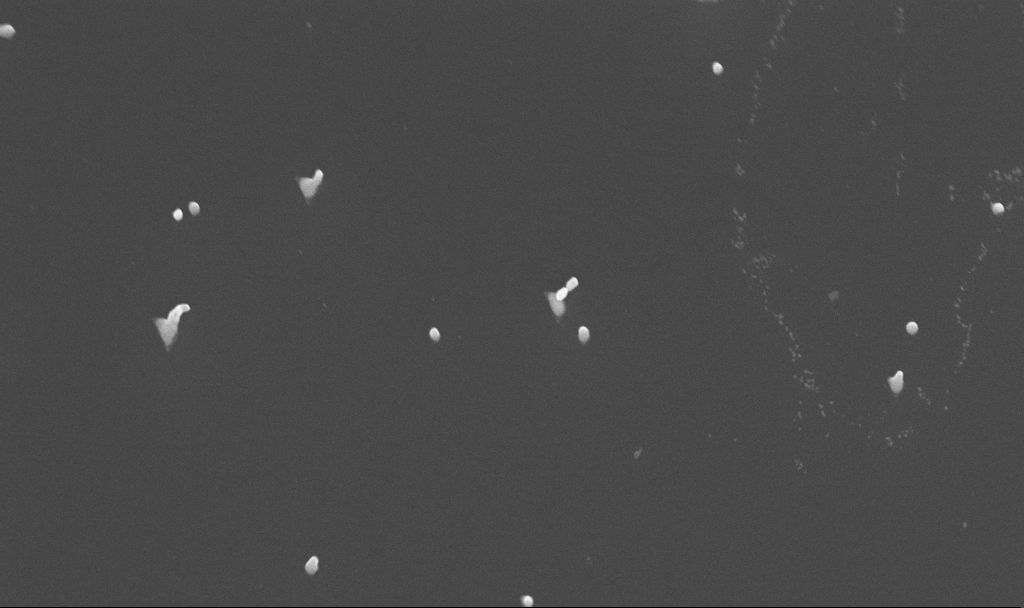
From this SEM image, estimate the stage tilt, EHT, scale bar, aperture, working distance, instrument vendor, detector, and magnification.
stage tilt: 45°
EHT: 10 kV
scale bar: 1000 nm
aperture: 30 µm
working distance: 4.8 mm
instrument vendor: Zeiss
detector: InLens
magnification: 50 K X